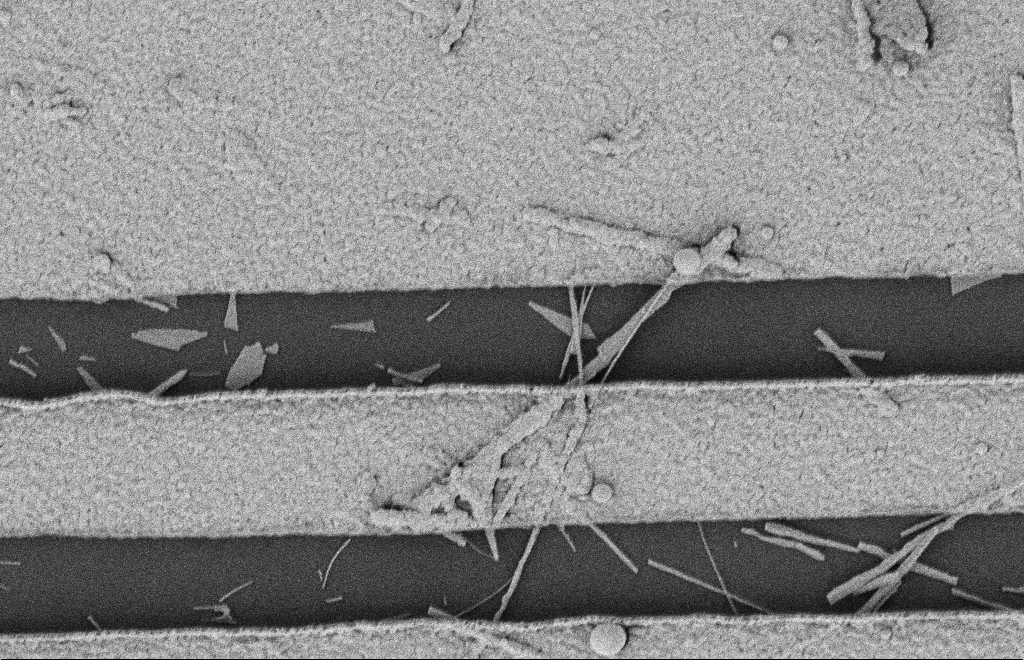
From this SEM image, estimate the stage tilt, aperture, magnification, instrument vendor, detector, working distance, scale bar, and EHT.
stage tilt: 0°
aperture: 20 µm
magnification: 21.1 K X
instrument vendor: Zeiss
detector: SE2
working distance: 12 mm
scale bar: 2000 nm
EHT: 2 kV